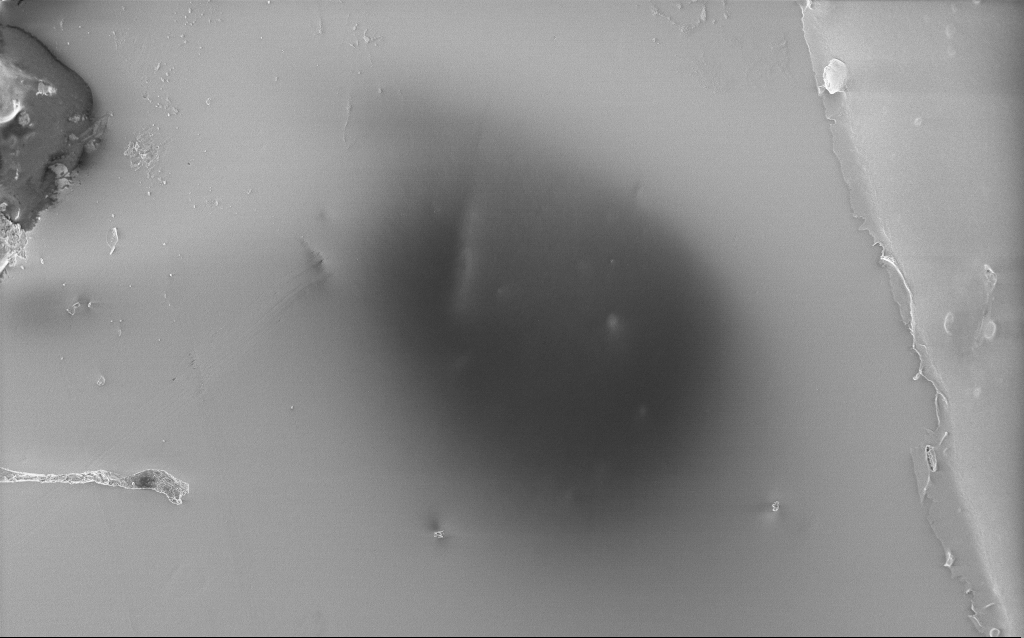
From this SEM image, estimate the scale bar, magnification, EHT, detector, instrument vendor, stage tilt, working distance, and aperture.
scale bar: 200000 nm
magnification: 0.235 K X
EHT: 3 kV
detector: InLens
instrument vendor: Zeiss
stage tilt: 0°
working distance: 2.7 mm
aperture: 30 µm